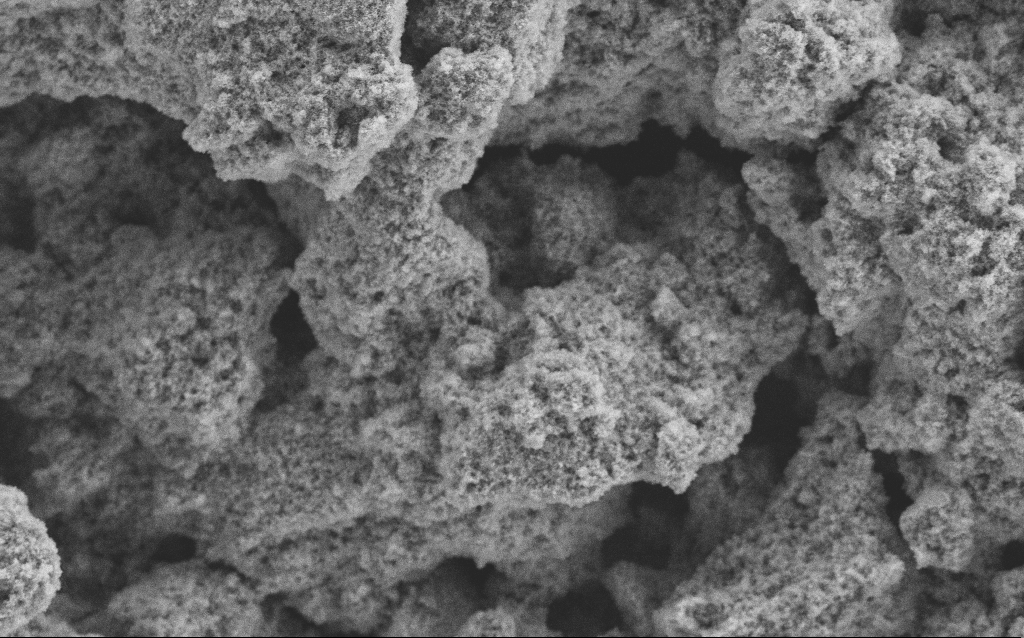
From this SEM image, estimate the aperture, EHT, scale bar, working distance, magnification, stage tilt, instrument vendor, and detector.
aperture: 30 µm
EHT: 5 kV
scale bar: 10000 nm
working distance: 4.1 mm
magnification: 6.41 K X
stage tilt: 0°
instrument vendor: Zeiss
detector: SE2